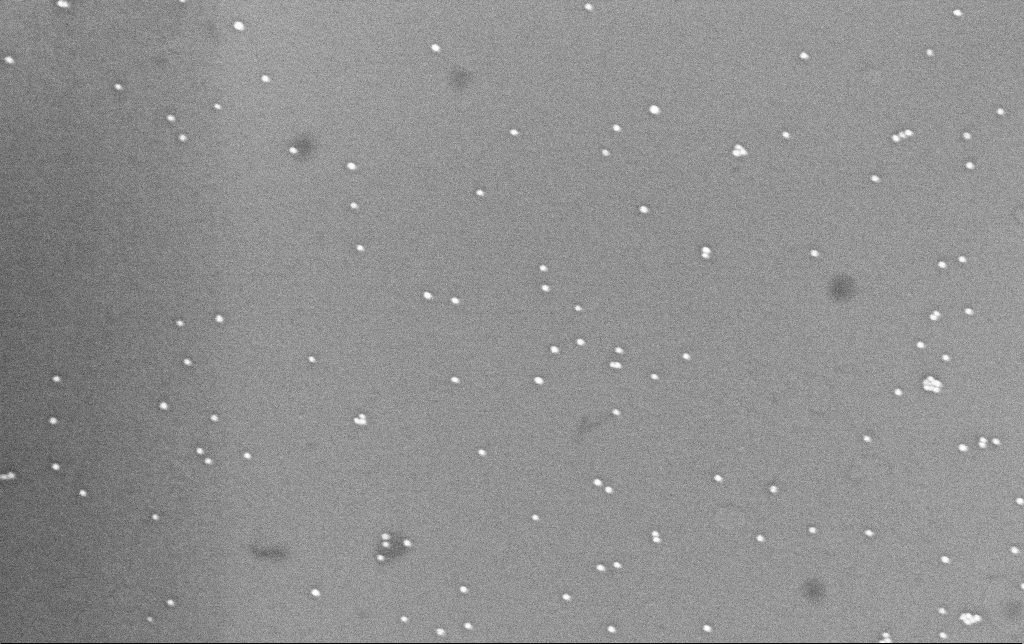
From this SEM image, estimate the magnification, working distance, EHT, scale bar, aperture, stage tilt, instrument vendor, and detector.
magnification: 124.83 K X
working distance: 6.6 mm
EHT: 8 kV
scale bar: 100 nm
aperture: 30 µm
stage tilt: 0°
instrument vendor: Zeiss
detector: InLens